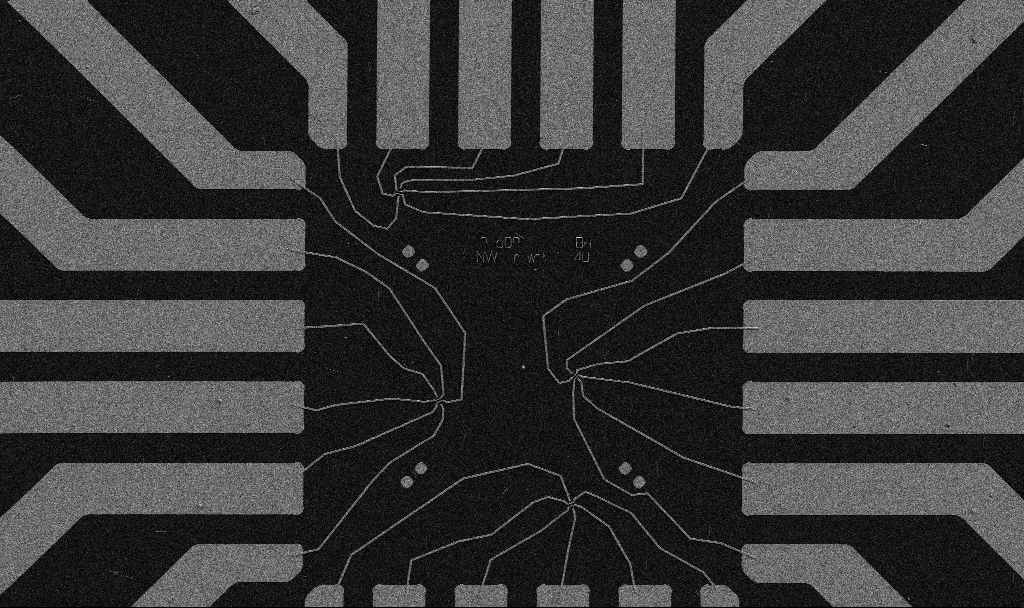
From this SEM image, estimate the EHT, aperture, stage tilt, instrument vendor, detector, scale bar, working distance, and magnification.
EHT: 5 kV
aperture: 30 µm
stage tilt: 0°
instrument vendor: Zeiss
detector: SE2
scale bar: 20000 nm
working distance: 10.7 mm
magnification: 1 K X